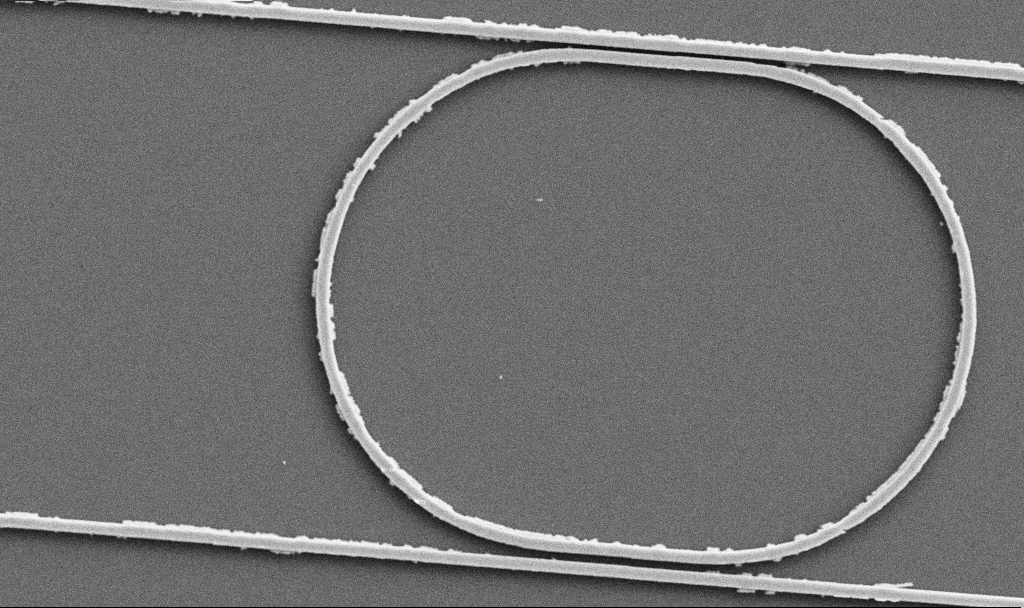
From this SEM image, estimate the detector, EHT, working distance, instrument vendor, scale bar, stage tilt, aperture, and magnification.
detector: SE2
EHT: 5 kV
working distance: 7.4 mm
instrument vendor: Zeiss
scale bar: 2000 nm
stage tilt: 0°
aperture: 30 µm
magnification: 9.34 K X